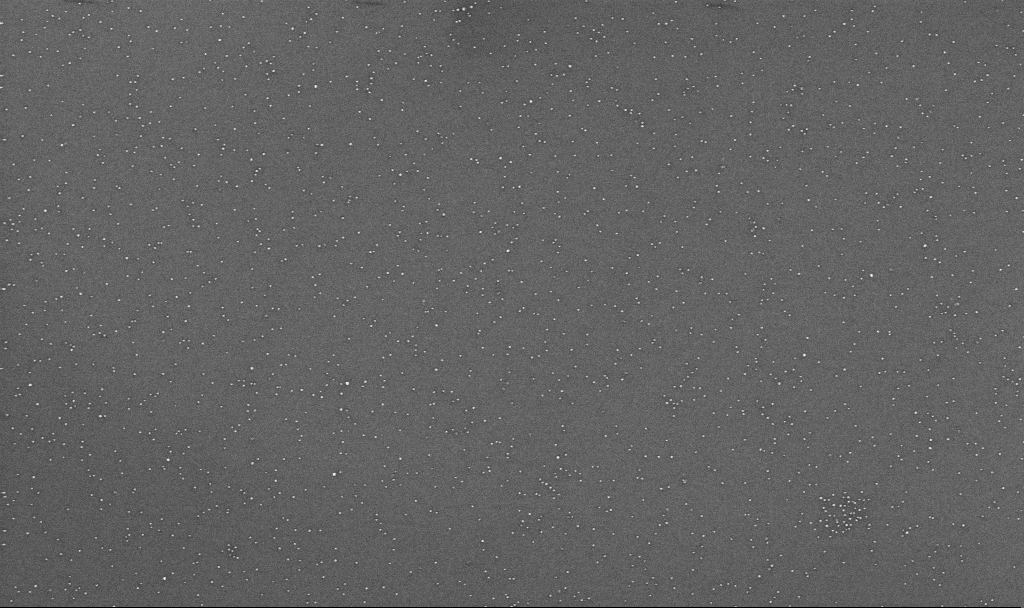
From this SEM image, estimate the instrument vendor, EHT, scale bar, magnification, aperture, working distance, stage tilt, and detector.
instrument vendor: Zeiss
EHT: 10 kV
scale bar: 1000 nm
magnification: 70 K X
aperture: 30 µm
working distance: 3.3 mm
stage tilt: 0°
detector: InLens